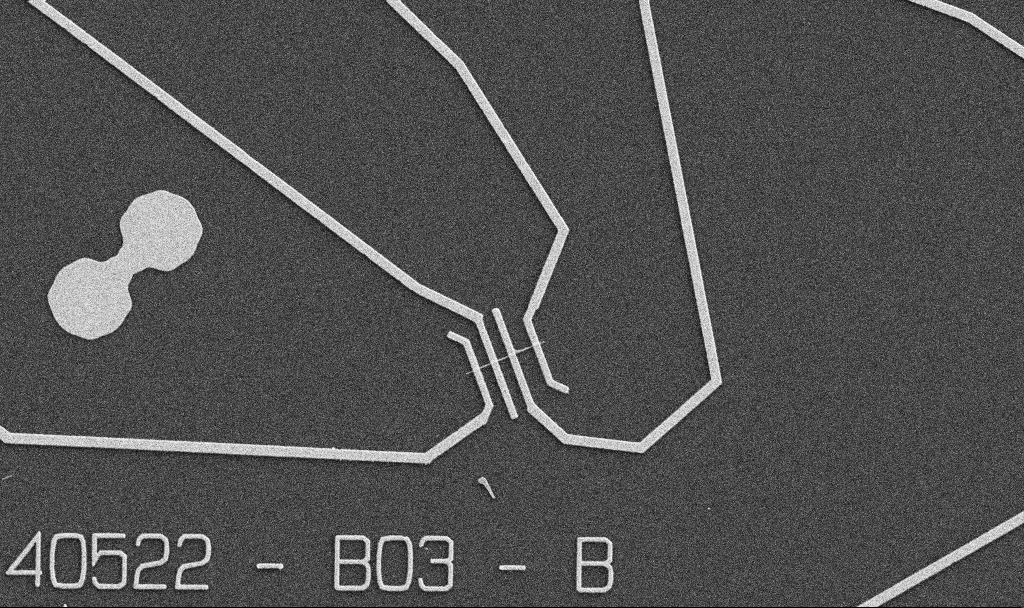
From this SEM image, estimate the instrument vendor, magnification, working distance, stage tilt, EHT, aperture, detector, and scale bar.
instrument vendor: Zeiss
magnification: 5 K X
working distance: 10.7 mm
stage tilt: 0°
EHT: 5 kV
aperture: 30 µm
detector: SE2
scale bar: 10000 nm